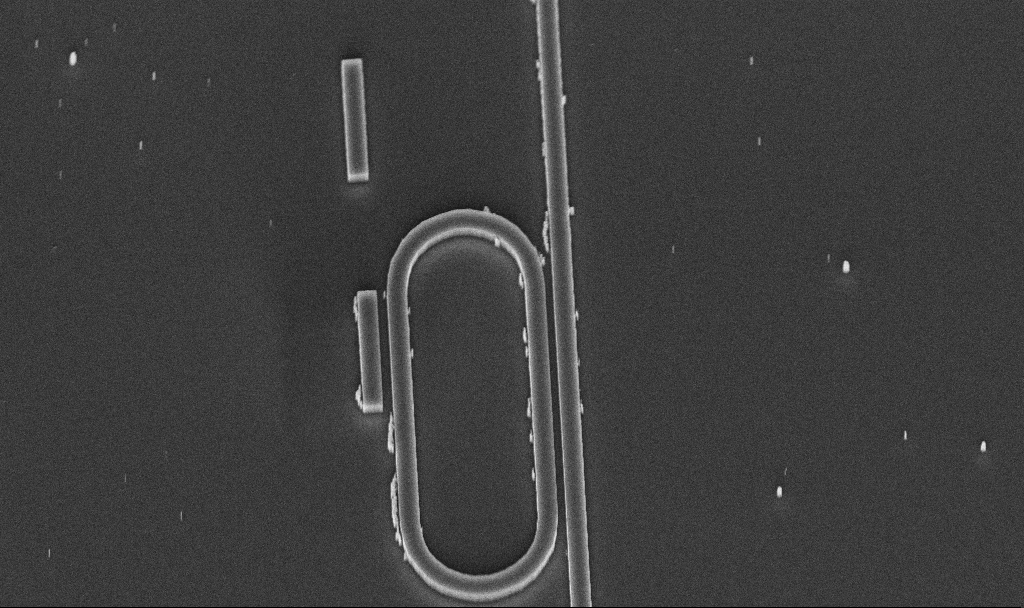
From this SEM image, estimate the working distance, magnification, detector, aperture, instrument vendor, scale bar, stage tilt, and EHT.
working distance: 9.8 mm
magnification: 14.46 K X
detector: InLens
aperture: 30 µm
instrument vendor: Zeiss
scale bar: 2000 nm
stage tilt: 45°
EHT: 5 kV